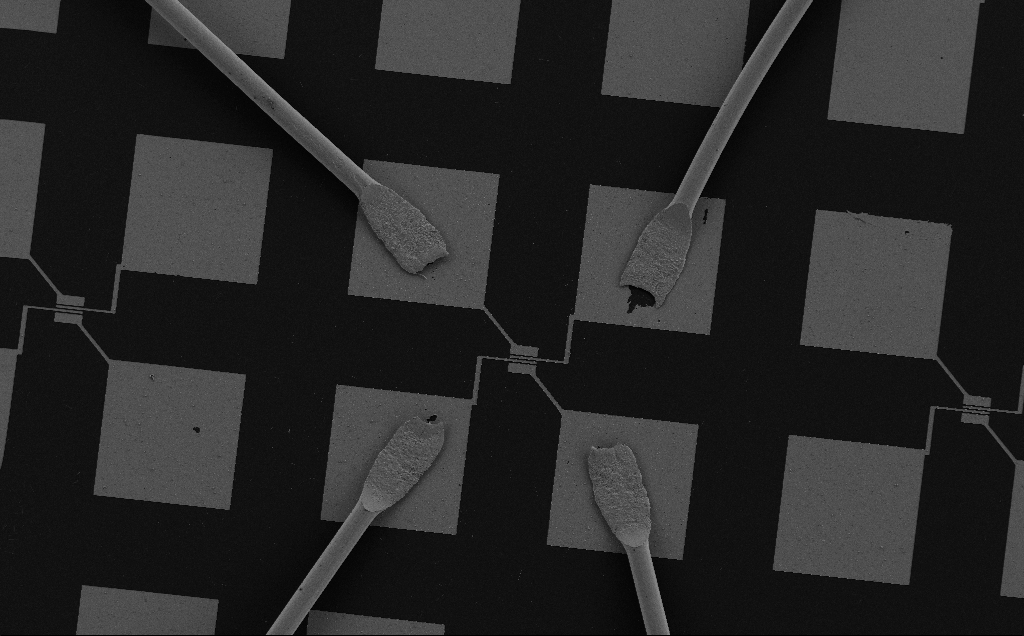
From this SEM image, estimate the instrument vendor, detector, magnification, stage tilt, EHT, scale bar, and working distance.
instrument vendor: Zeiss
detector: SE2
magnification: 0.339 K X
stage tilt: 0°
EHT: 5 kV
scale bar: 200000 nm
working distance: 6 mm